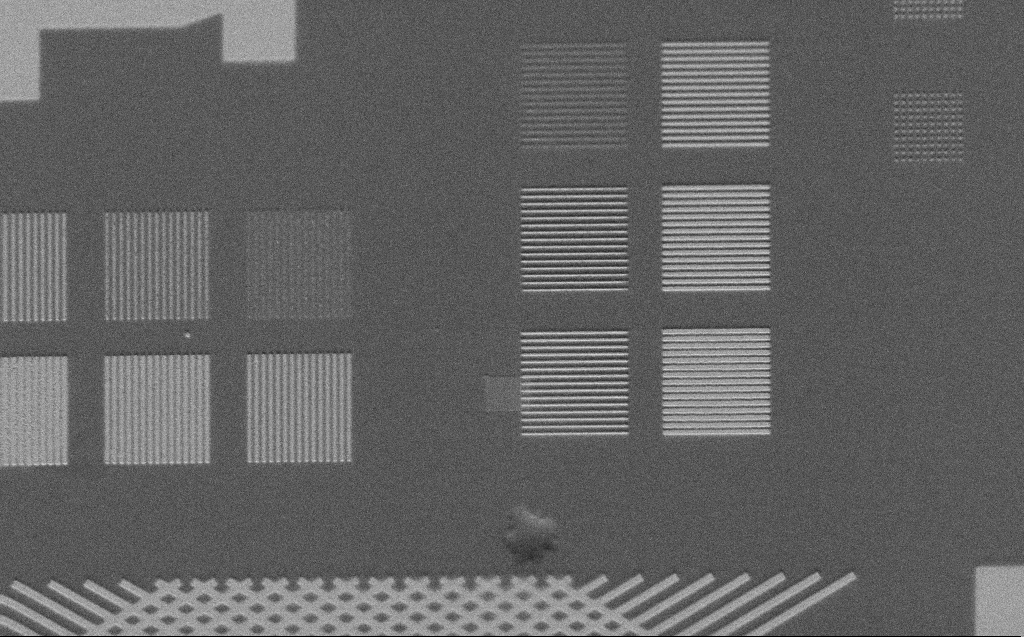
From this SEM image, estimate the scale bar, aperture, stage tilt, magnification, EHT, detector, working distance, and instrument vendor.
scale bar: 10000 nm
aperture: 30 µm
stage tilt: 45°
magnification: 2.63 K X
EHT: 3 kV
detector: SE2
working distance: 5 mm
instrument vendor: Zeiss